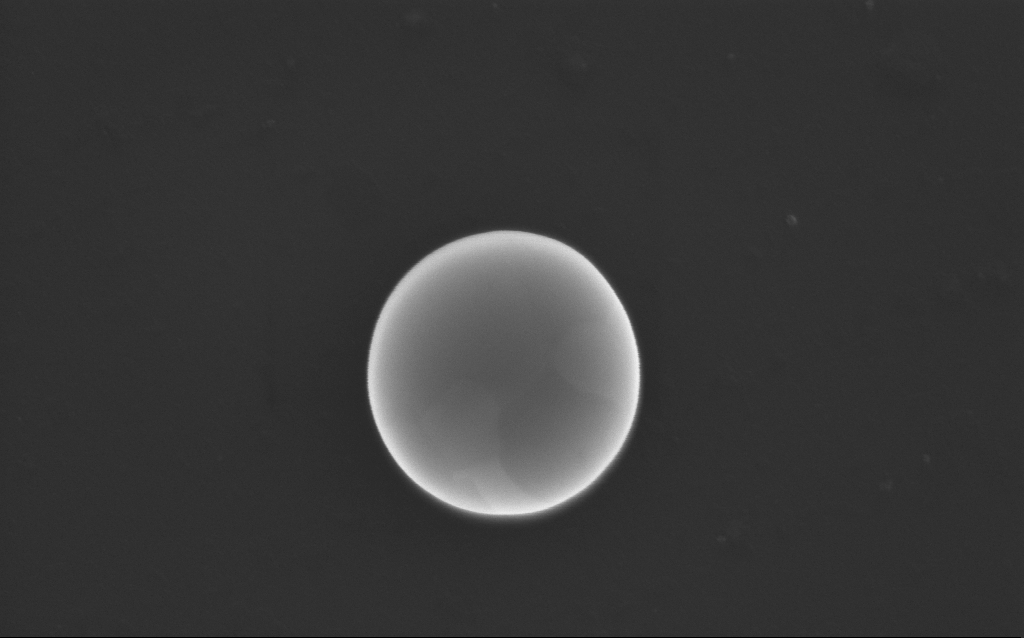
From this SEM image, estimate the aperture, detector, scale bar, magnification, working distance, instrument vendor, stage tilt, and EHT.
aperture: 30 µm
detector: InLens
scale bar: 200 nm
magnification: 100.06 K X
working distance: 3 mm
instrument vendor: Zeiss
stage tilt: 0°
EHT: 10 kV